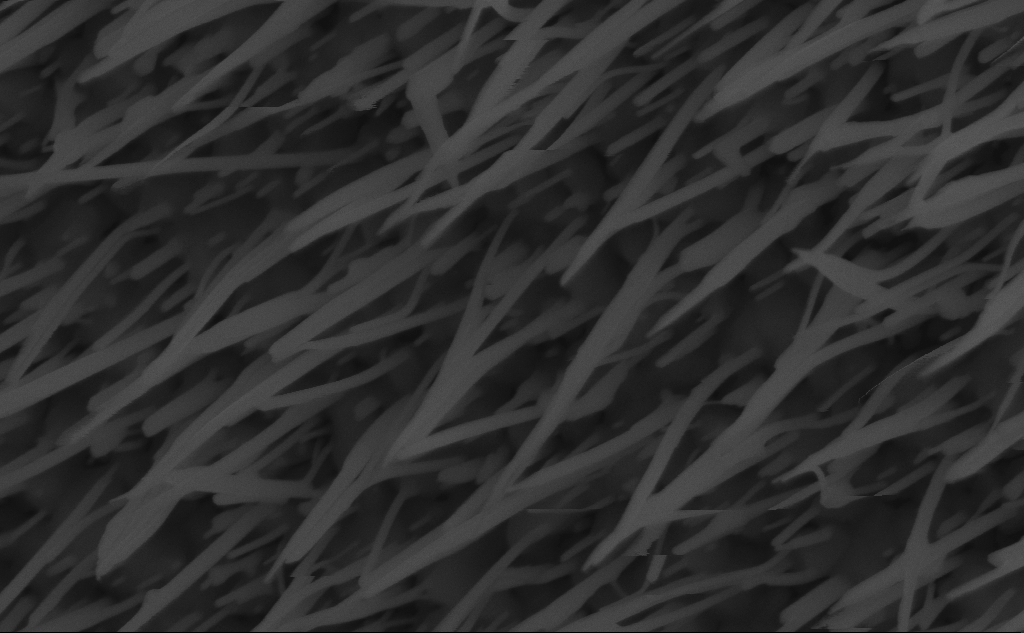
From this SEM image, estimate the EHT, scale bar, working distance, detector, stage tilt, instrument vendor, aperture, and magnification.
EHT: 10 kV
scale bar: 200 nm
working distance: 6 mm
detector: InLens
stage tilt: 45°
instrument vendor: Zeiss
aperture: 30 µm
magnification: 111.72 K X